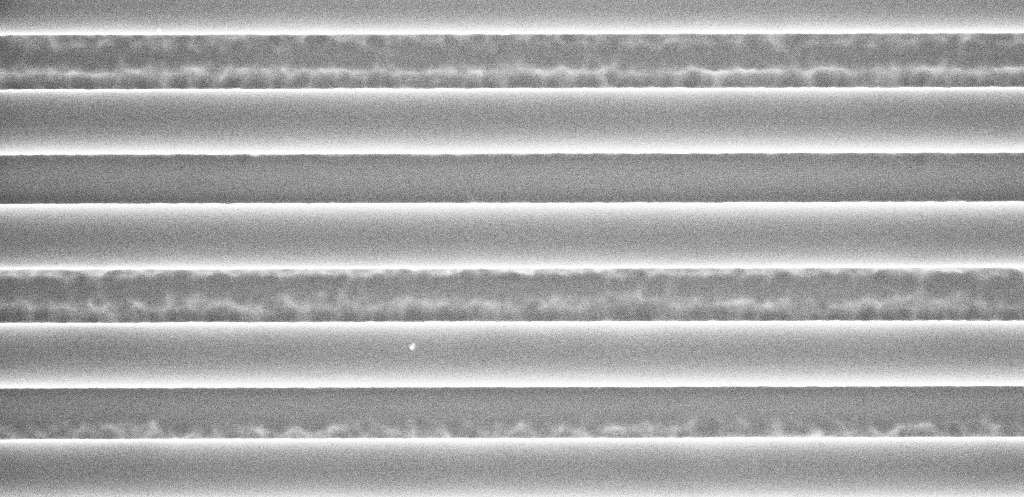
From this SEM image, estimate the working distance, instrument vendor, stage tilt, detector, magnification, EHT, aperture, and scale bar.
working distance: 10.1 mm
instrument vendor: Zeiss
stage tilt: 0°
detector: InLens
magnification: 66.45 K X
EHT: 5 kV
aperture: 30 µm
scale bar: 1000 nm